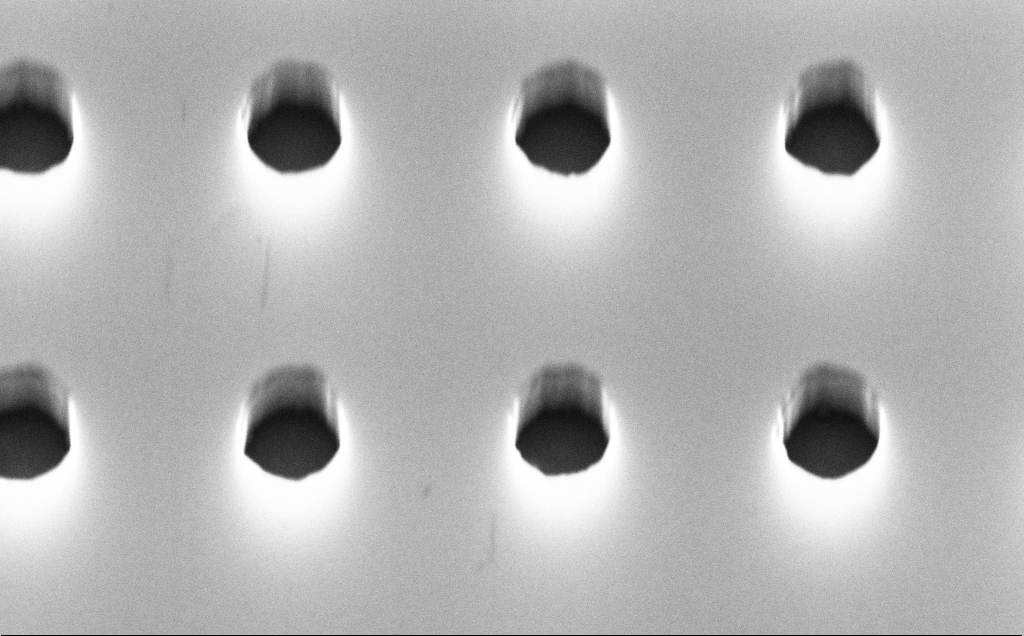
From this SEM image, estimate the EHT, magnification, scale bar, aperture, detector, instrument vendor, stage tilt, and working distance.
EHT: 10 kV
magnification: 94.31 K X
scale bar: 200 nm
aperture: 30 µm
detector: InLens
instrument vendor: Zeiss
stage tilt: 45°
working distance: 4 mm